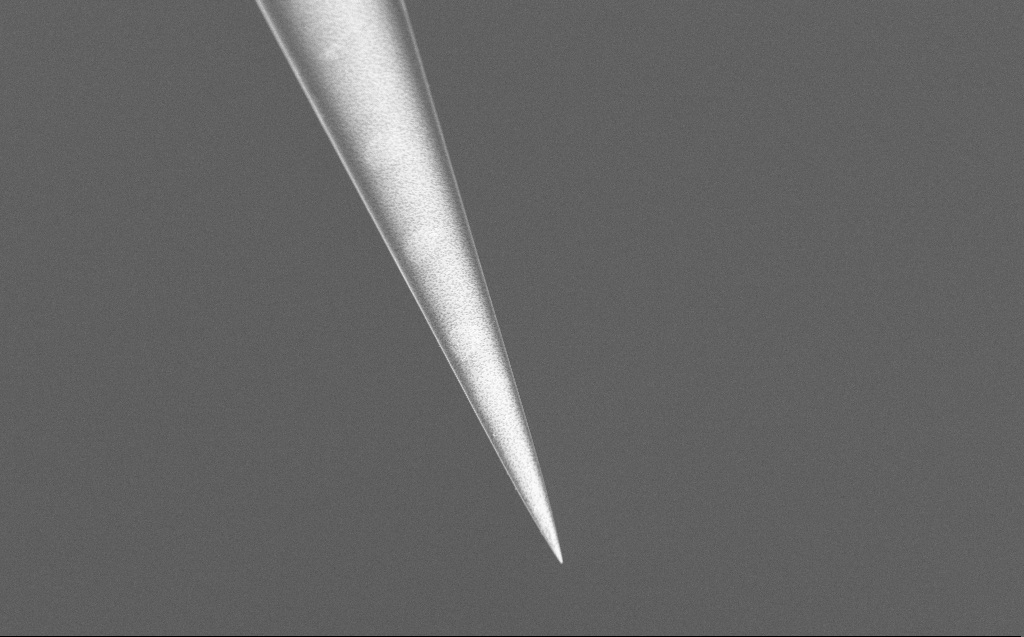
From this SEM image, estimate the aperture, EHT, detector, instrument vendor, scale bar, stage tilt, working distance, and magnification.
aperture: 30 µm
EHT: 5 kV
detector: InLens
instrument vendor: Zeiss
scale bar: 10000 nm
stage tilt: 45°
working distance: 7 mm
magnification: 5 K X